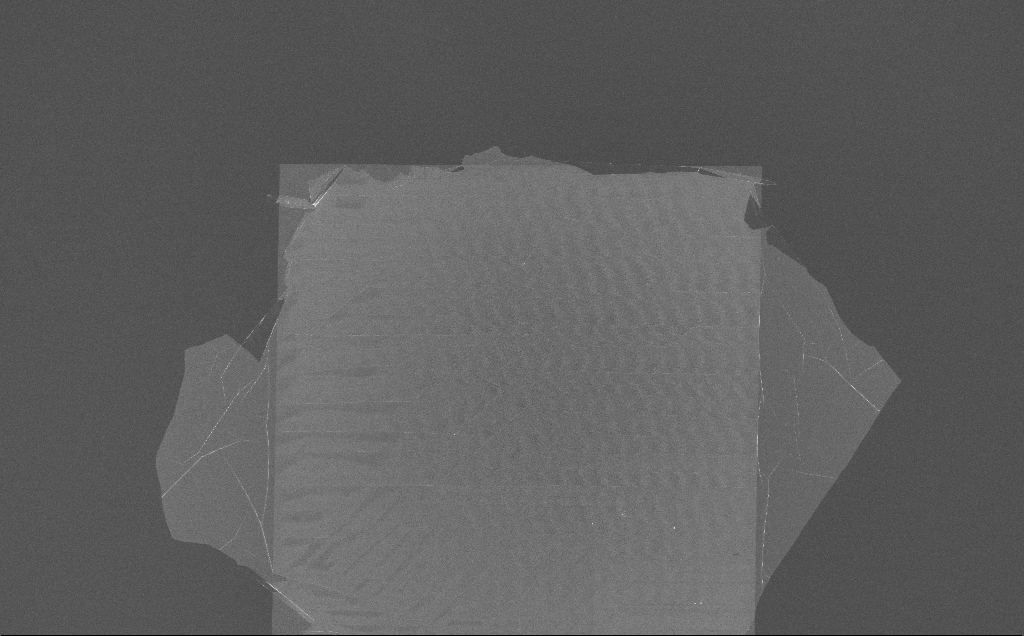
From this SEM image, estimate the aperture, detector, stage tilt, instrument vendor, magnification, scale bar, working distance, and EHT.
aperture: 30 µm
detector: InLens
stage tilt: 0°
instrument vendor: Zeiss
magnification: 0.239 K X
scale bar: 100000 nm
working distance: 7 mm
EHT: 10 kV